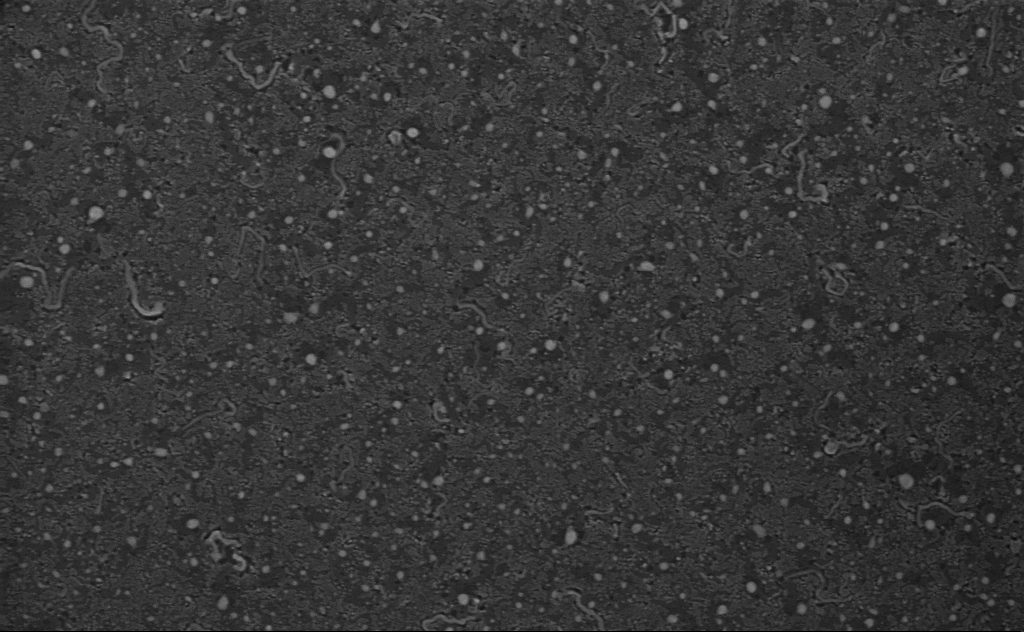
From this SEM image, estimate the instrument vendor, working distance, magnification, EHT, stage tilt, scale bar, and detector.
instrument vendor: Zeiss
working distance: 4 mm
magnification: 80 K X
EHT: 3 kV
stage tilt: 0°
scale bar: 200 nm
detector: InLens